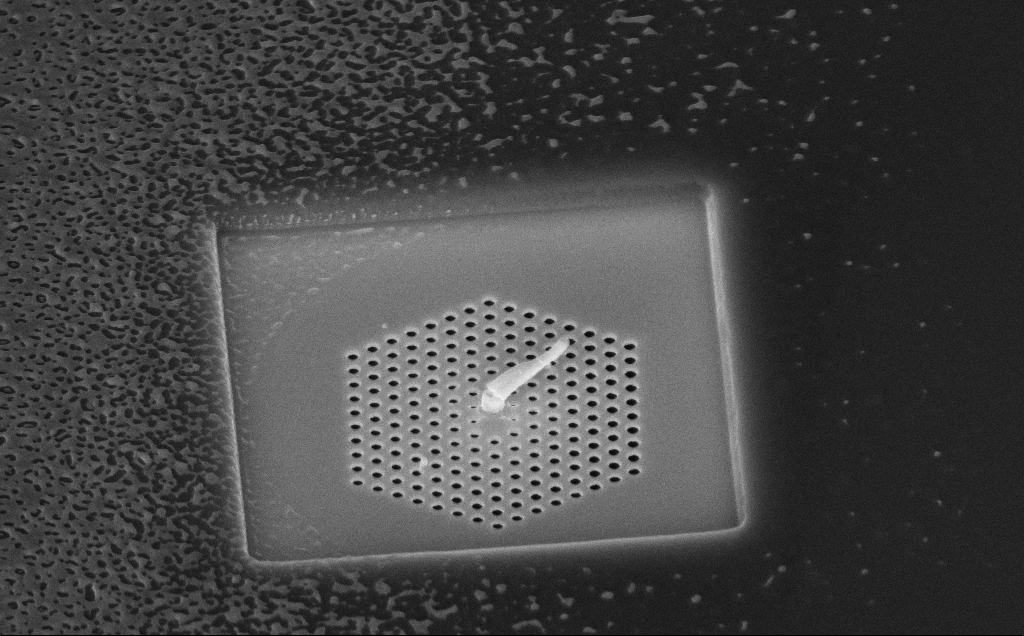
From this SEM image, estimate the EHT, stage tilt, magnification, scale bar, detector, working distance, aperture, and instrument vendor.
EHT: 10 kV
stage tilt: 45°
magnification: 29.22 K X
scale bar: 2000 nm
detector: InLens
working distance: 11 mm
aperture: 30 µm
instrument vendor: Zeiss